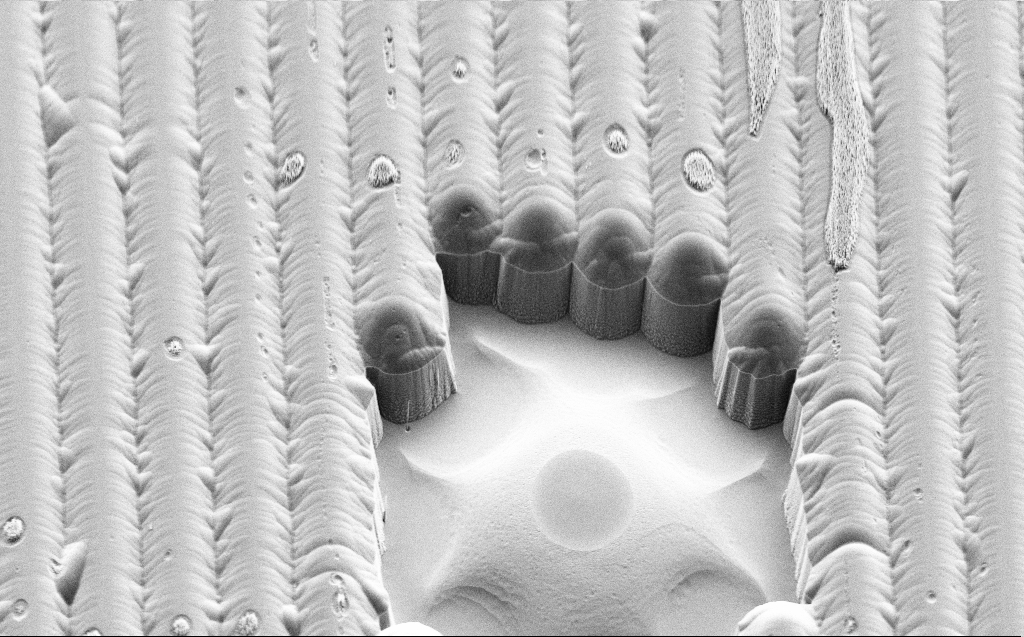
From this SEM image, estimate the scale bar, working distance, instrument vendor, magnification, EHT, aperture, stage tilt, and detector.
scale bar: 20000 nm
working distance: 8 mm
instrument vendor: Zeiss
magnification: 2.76 K X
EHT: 3 kV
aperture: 30 µm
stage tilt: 45°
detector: SE2